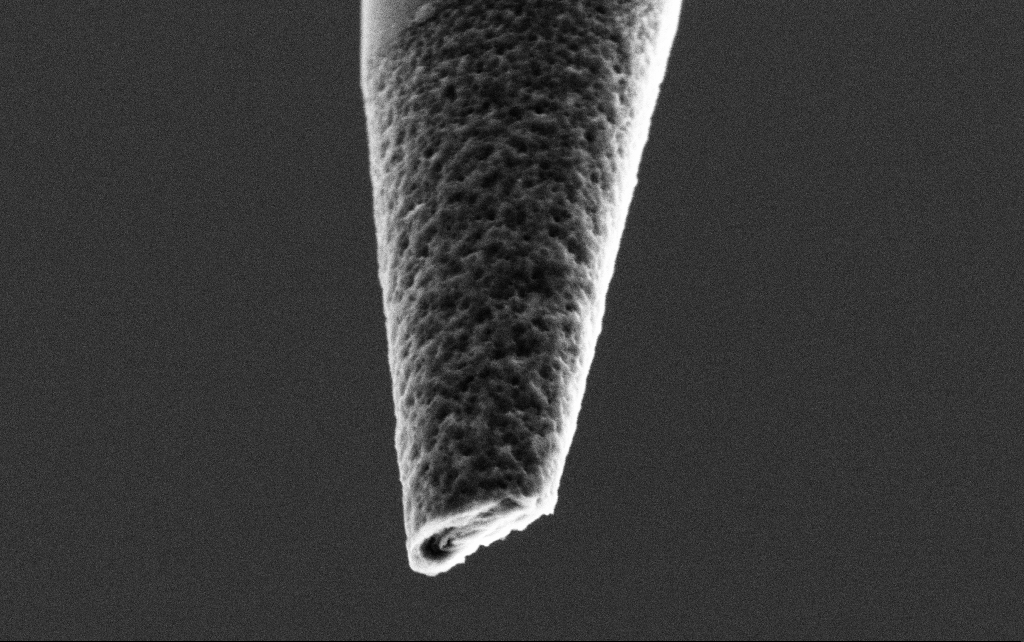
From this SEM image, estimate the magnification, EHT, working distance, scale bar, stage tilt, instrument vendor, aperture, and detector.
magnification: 50 K X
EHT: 3 kV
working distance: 7.7 mm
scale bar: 1000 nm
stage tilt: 45°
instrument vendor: Zeiss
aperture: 30 µm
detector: SE2